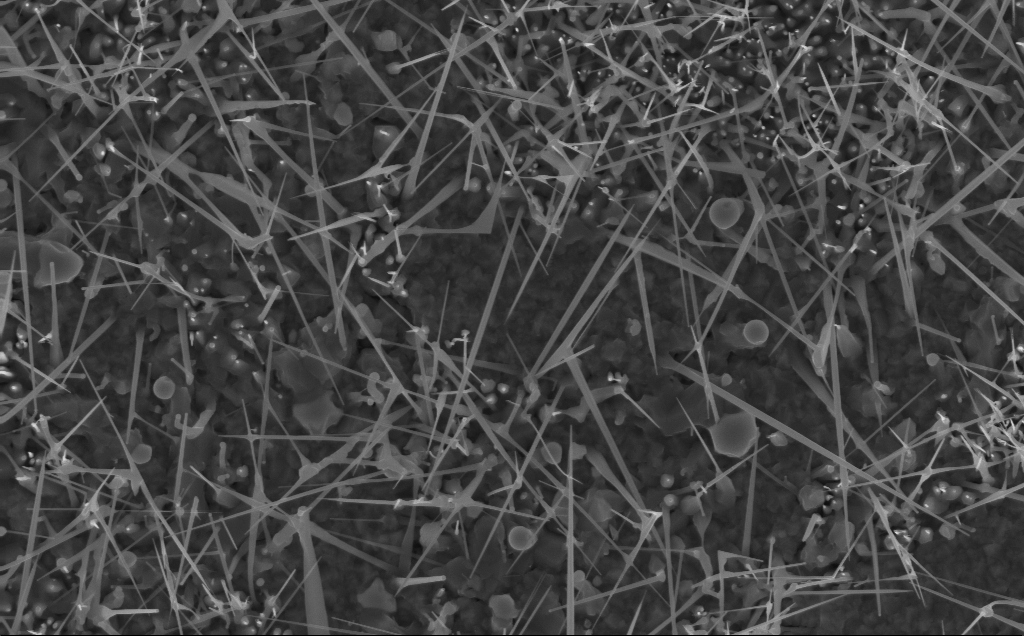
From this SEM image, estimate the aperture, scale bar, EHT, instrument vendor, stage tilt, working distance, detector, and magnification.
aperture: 30 µm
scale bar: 1000 nm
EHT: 10 kV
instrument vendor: Zeiss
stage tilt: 0°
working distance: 3 mm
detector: InLens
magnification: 20 K X